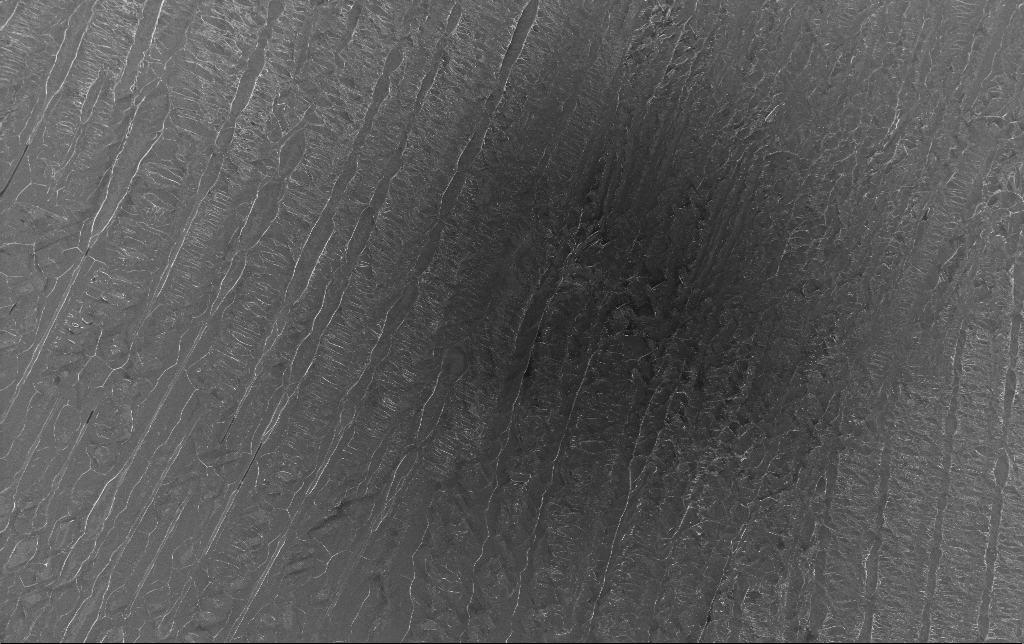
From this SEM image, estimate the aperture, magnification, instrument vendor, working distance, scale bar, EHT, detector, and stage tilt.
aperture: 30 µm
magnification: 0.316 K X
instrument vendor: Zeiss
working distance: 3.1 mm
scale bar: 100000 nm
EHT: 5 kV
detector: InLens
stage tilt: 0°